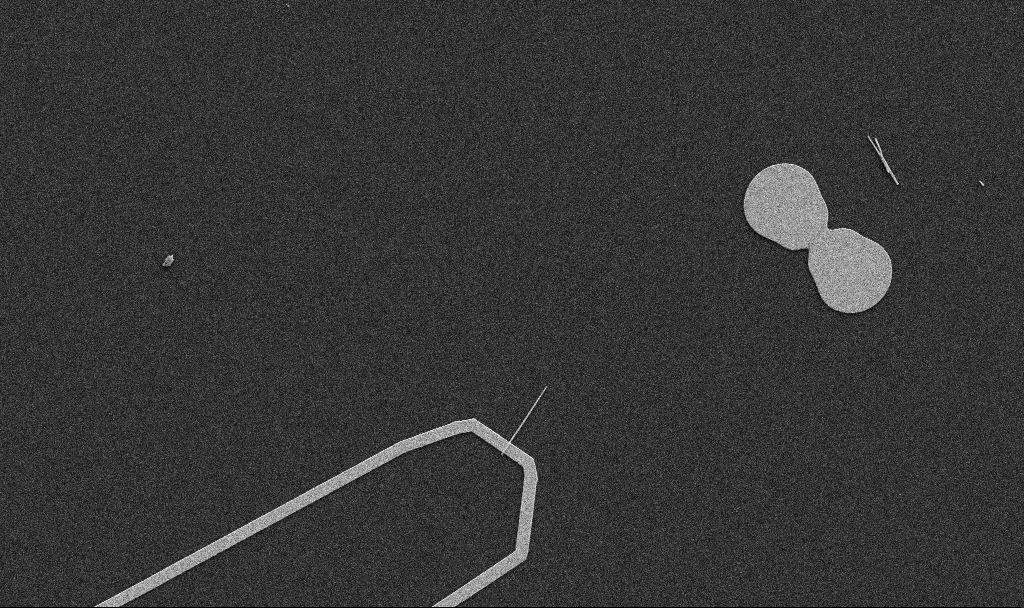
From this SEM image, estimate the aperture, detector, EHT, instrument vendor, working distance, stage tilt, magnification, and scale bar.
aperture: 30 µm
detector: SE2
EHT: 5 kV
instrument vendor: Zeiss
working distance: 10.7 mm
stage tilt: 0°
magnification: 5 K X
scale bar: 10000 nm